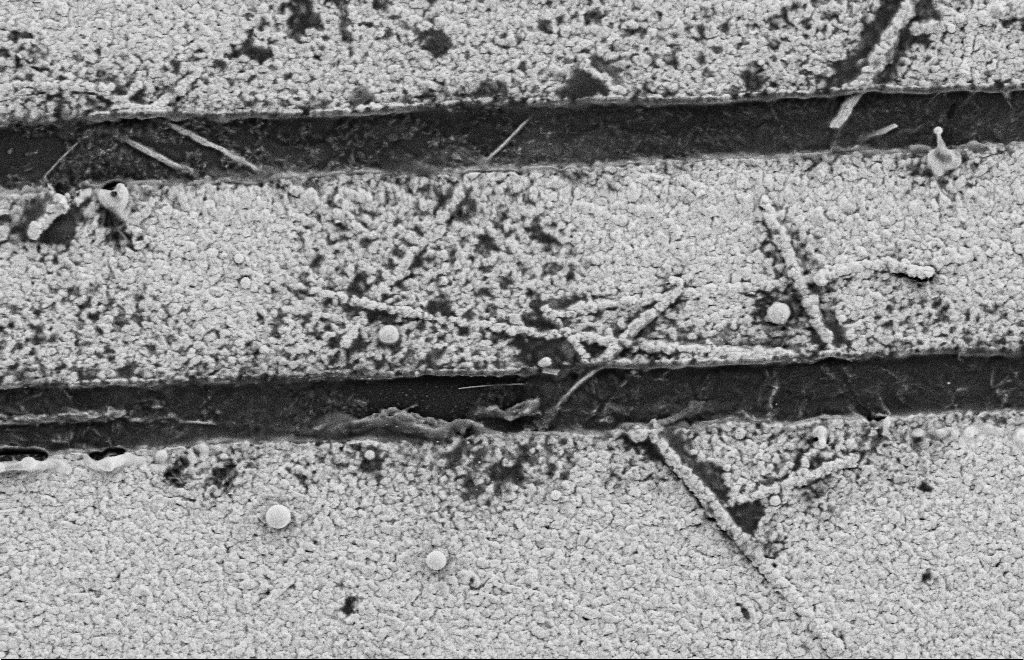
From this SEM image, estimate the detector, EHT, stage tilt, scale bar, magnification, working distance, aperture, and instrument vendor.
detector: SE2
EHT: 2 kV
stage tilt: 0°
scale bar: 1000 nm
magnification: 24.25 K X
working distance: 9 mm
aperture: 20 µm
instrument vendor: Zeiss